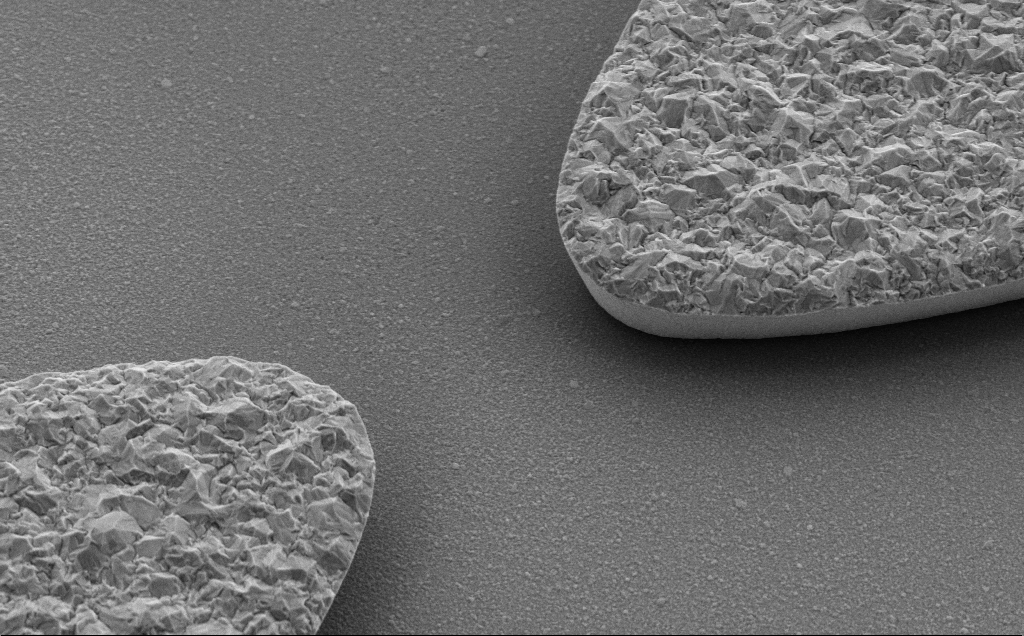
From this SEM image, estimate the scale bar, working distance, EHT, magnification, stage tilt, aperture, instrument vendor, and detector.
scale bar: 2000 nm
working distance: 9 mm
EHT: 5 kV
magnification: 19.98 K X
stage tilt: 30°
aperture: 30 µm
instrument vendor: Zeiss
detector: SE2